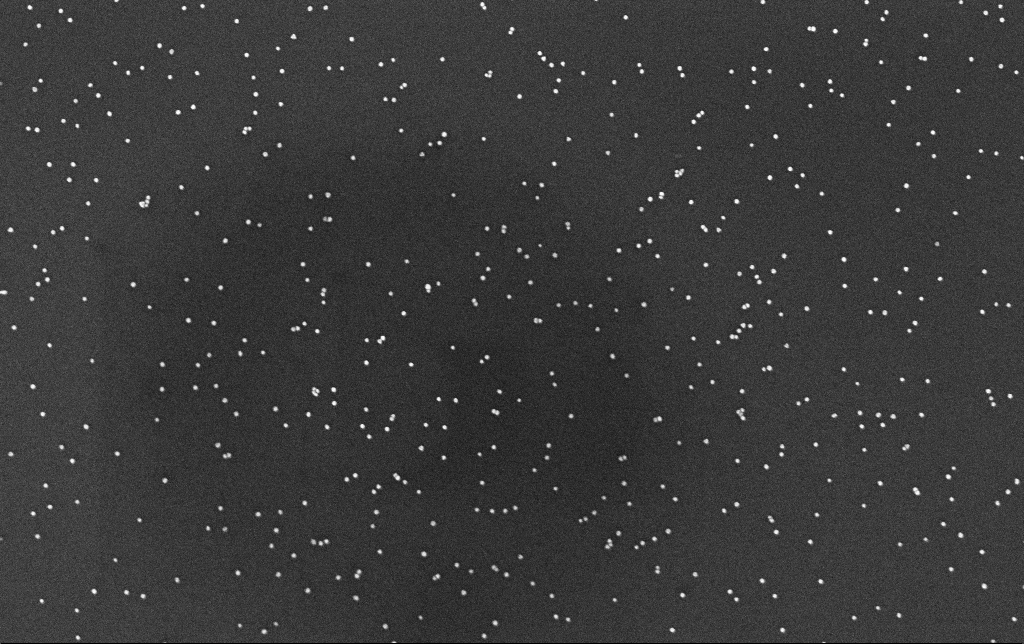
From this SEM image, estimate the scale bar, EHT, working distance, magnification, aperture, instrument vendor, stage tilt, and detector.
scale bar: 200 nm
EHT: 10 kV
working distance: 3.4 mm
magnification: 100 K X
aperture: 30 µm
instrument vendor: Zeiss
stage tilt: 0°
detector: InLens